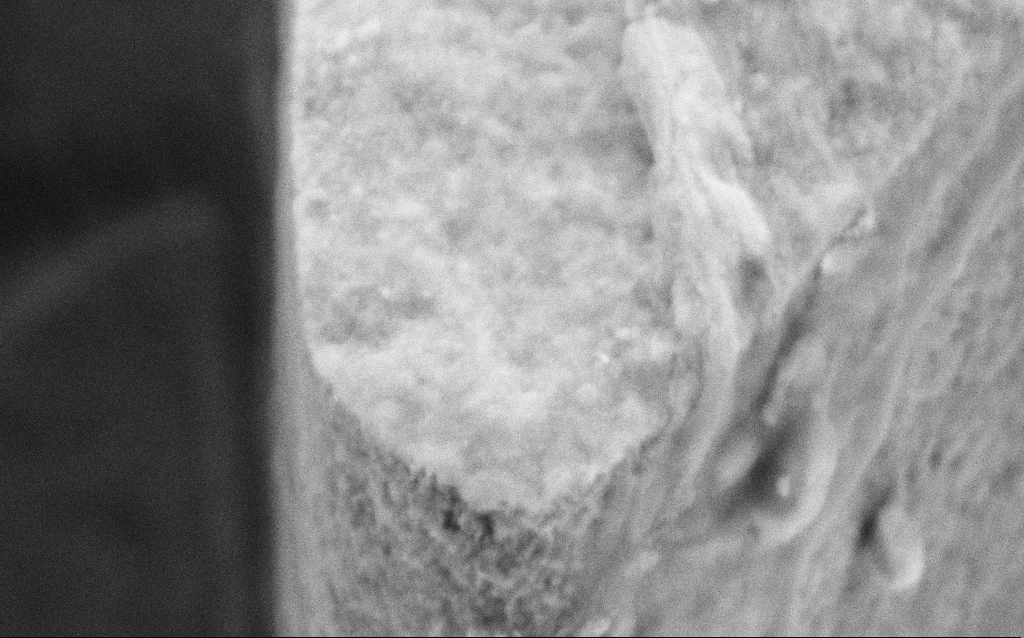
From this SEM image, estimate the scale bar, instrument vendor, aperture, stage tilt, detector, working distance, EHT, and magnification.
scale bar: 200 nm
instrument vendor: Zeiss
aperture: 30 µm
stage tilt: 45°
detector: SE2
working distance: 6 mm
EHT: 5 kV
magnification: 85.03 K X